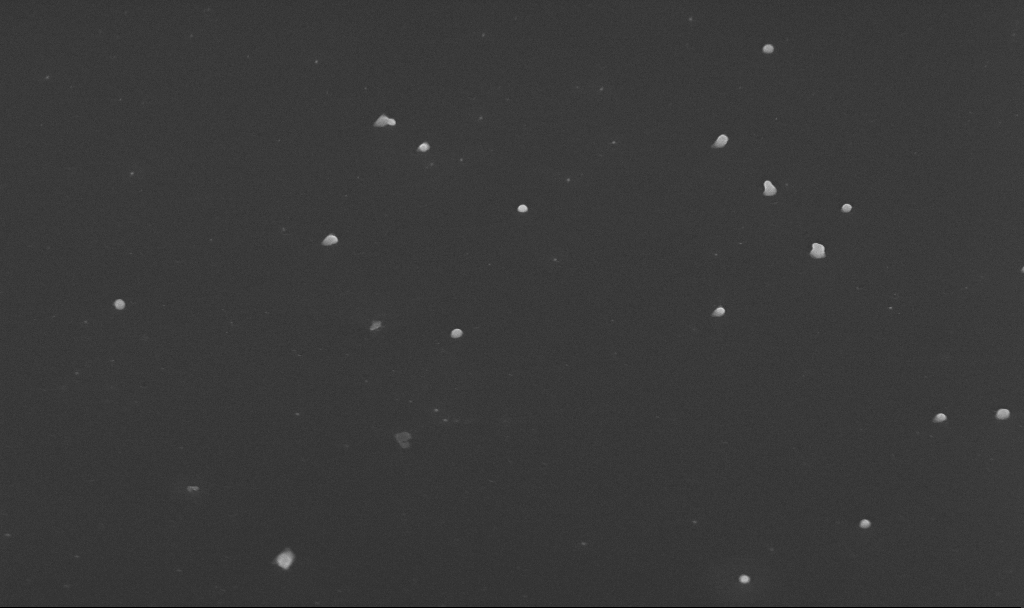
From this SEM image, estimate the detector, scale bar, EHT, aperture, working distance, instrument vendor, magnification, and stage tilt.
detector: InLens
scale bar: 1000 nm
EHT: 10 kV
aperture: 30 µm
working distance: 5.6 mm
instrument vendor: Zeiss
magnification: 50 K X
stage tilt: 45°